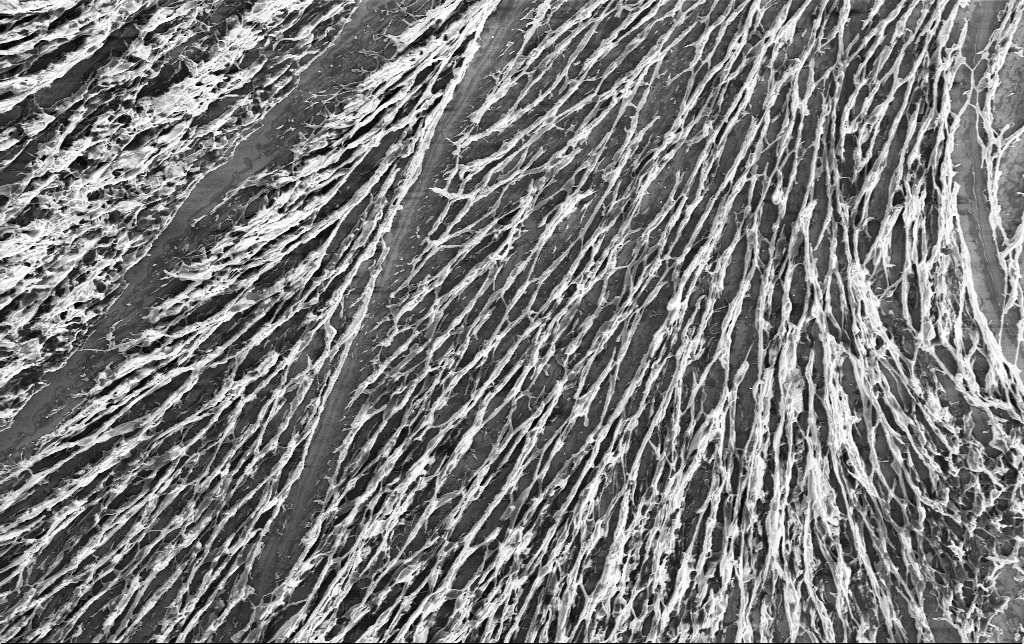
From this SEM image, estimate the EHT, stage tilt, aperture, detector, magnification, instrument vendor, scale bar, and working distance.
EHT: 3 kV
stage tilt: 0°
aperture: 30 µm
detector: InLens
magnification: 2.97 K X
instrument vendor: Zeiss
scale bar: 10000 nm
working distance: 3.2 mm